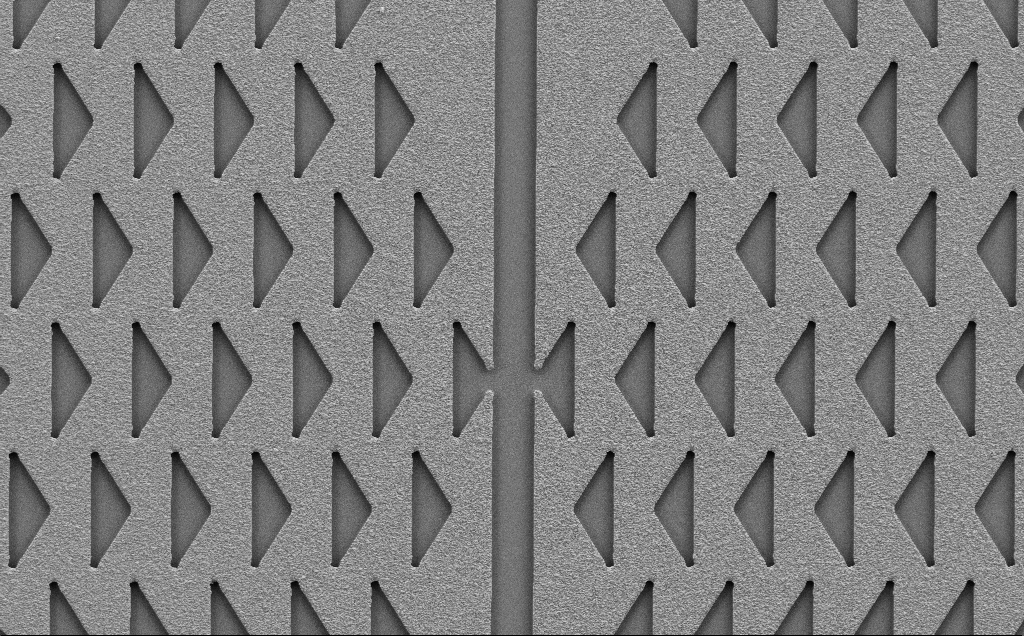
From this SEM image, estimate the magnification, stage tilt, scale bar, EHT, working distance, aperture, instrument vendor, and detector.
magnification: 1.24 K X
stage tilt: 0°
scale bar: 10000 nm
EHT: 5 kV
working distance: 6 mm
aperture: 30 µm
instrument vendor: Zeiss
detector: SE2